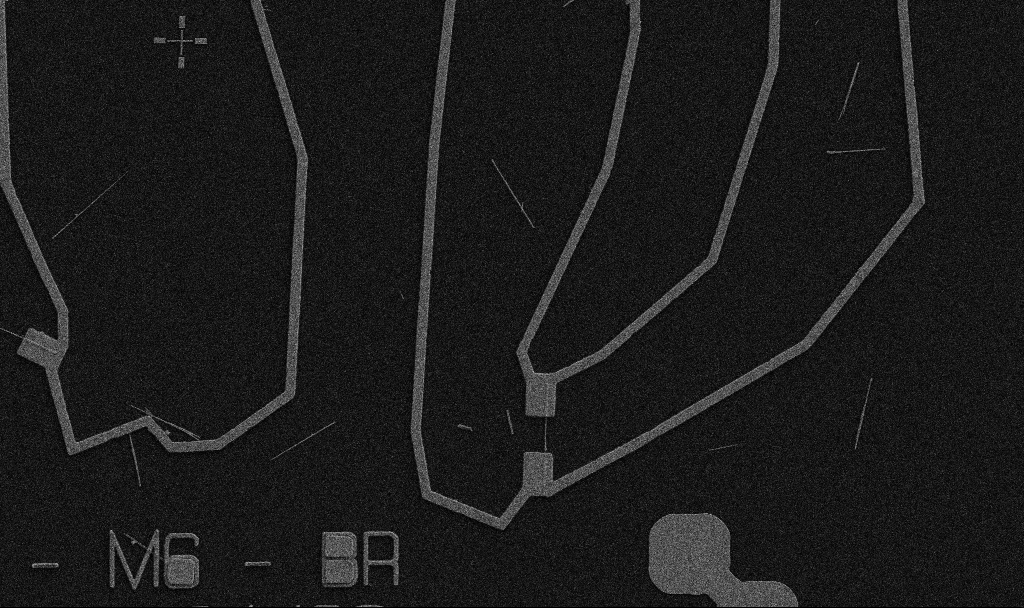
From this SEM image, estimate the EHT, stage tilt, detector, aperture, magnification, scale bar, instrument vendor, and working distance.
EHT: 5 kV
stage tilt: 0°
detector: SE2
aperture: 30 µm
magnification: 5 K X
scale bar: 10000 nm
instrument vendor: Zeiss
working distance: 10.7 mm